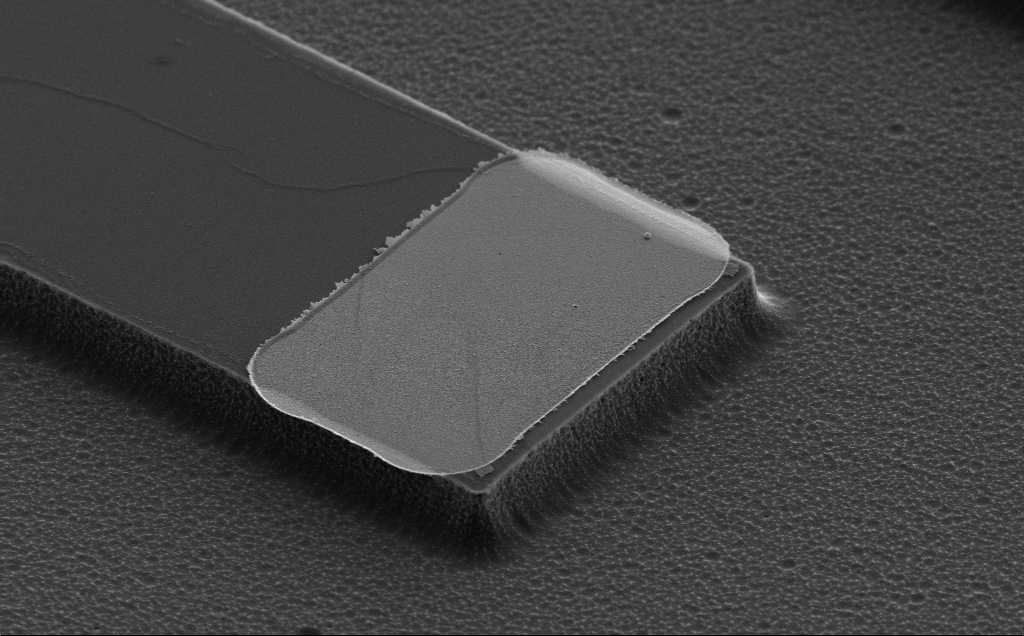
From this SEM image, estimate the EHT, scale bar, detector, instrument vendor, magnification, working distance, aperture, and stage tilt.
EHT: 5 kV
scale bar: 2000 nm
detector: SE2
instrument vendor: Zeiss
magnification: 9.22 K X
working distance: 10 mm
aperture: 30 µm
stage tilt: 50°